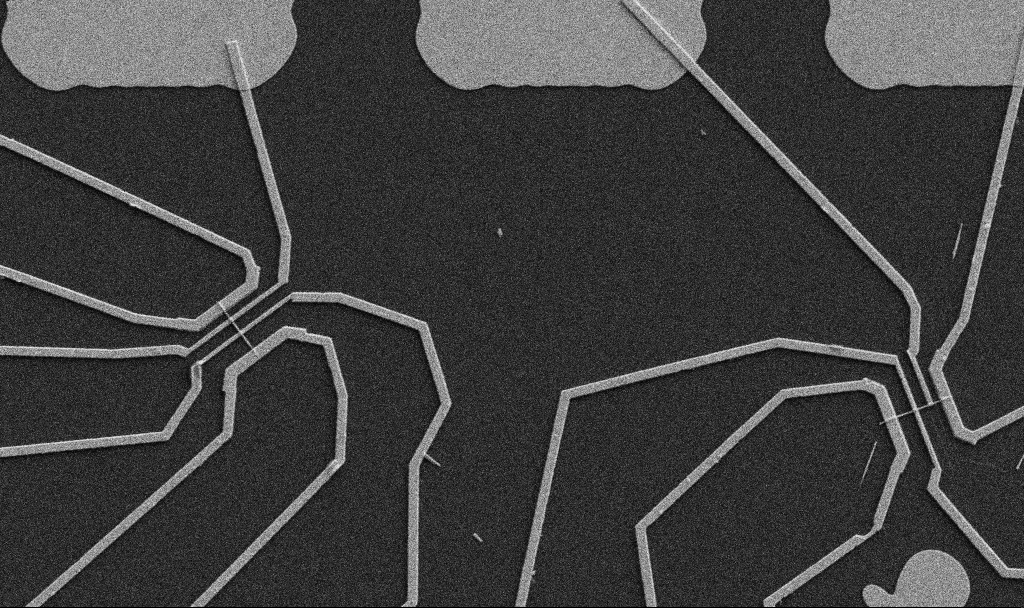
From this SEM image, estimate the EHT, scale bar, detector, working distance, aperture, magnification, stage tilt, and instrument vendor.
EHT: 5 kV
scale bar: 10000 nm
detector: SE2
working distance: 10.7 mm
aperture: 30 µm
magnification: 5 K X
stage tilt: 0°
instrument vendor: Zeiss